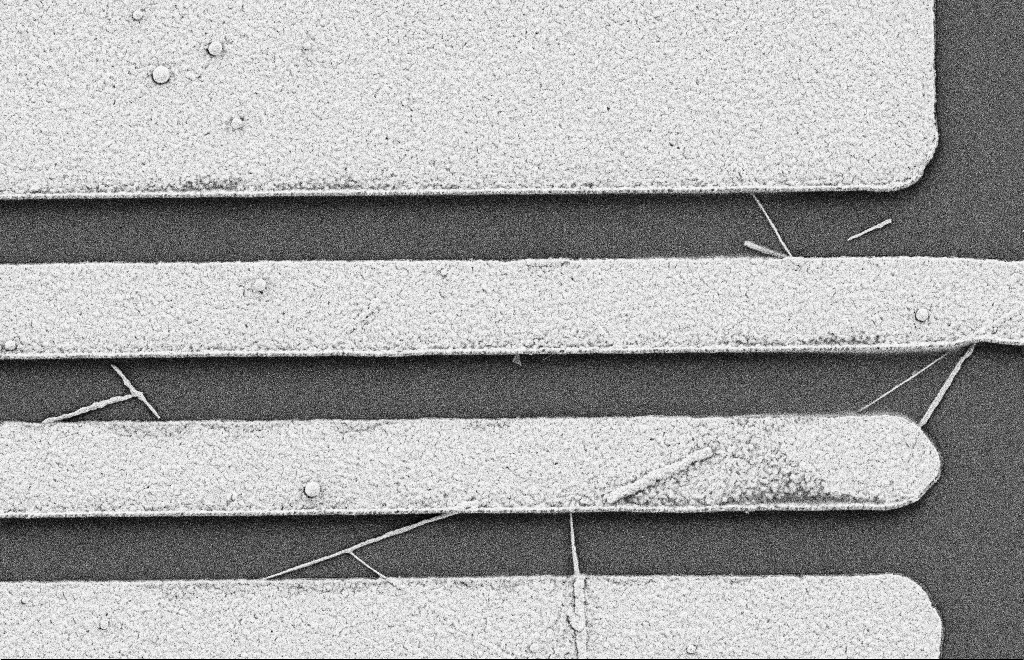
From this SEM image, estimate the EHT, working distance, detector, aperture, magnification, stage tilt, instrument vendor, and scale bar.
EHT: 2 kV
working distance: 10 mm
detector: SE2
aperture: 20 µm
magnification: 14.69 K X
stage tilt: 0°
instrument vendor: Zeiss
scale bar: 2000 nm